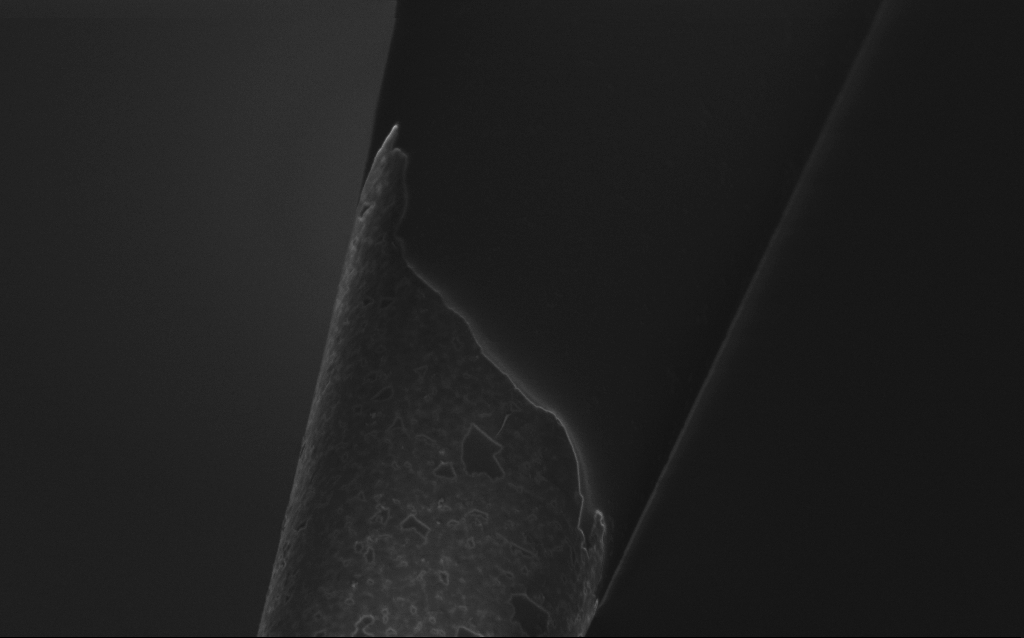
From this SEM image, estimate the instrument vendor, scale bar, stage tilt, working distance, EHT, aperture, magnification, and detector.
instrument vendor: Zeiss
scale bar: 2000 nm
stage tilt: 45°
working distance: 6 mm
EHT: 1 kV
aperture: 30 µm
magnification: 25 K X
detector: InLens